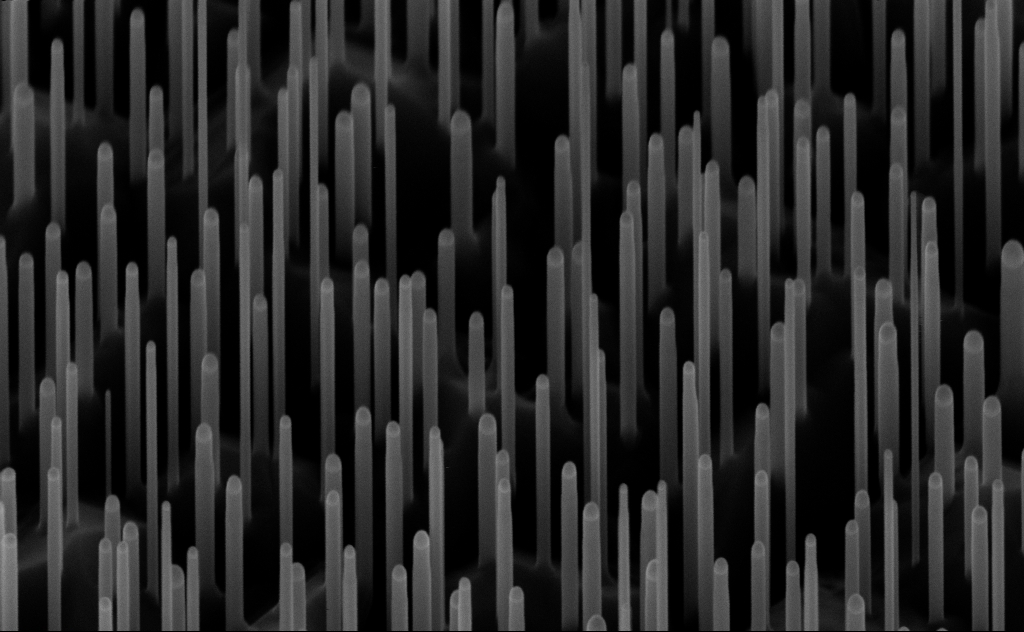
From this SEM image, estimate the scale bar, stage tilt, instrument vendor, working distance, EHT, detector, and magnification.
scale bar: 200 nm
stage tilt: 45°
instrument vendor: Zeiss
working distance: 7 mm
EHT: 10 kV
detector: InLens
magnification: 80 K X